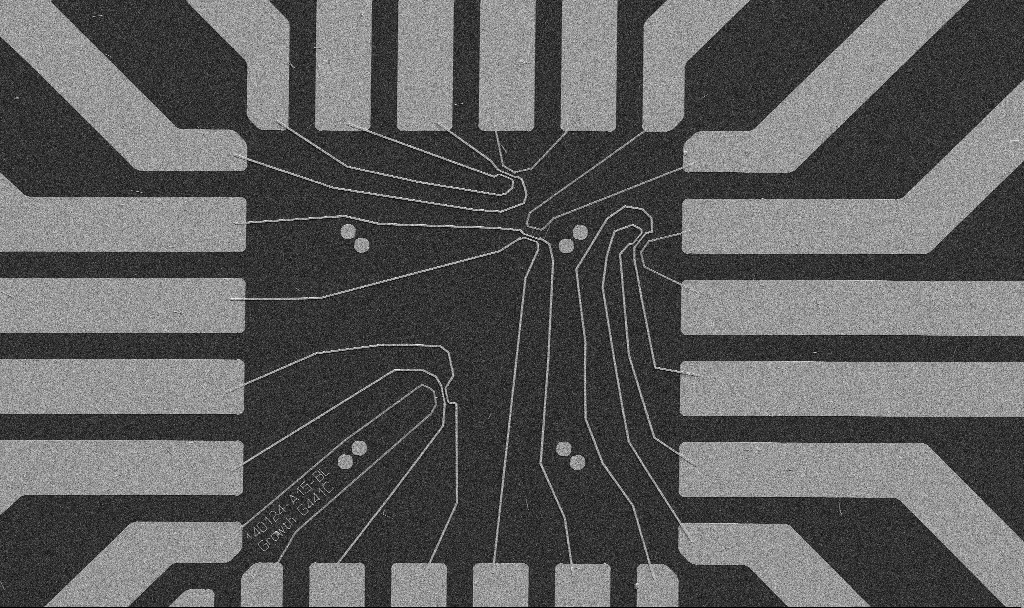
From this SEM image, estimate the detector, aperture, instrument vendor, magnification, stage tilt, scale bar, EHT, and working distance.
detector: SE2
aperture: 30 µm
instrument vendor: Zeiss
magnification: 1 K X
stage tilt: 0°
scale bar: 20000 nm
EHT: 5 kV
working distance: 10.7 mm